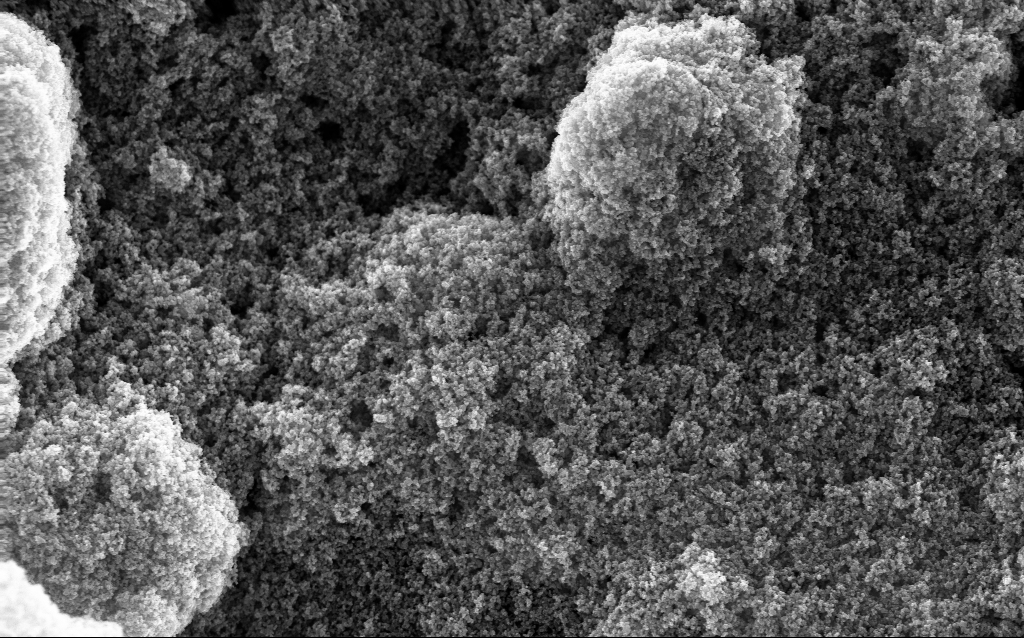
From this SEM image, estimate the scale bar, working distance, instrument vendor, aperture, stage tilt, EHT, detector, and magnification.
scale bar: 1000 nm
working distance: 2.7 mm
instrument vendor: Zeiss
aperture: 30 µm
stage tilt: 0°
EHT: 10 kV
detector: InLens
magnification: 37.88 K X